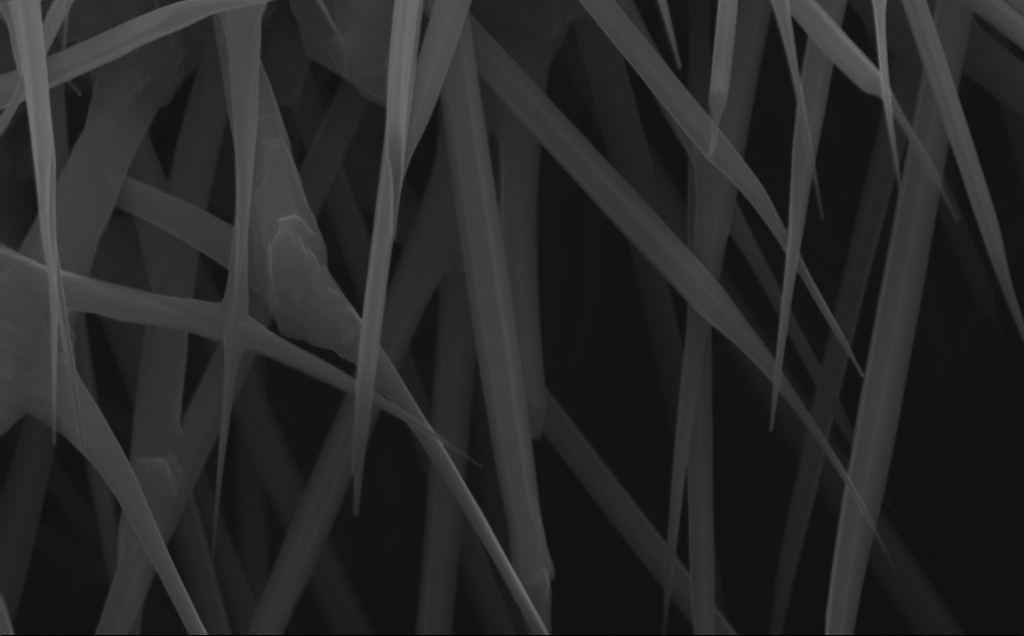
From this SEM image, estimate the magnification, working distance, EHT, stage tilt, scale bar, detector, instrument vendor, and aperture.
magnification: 85.25 K X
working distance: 7 mm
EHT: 10 kV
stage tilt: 0°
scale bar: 200 nm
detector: InLens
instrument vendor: Zeiss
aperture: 30 µm